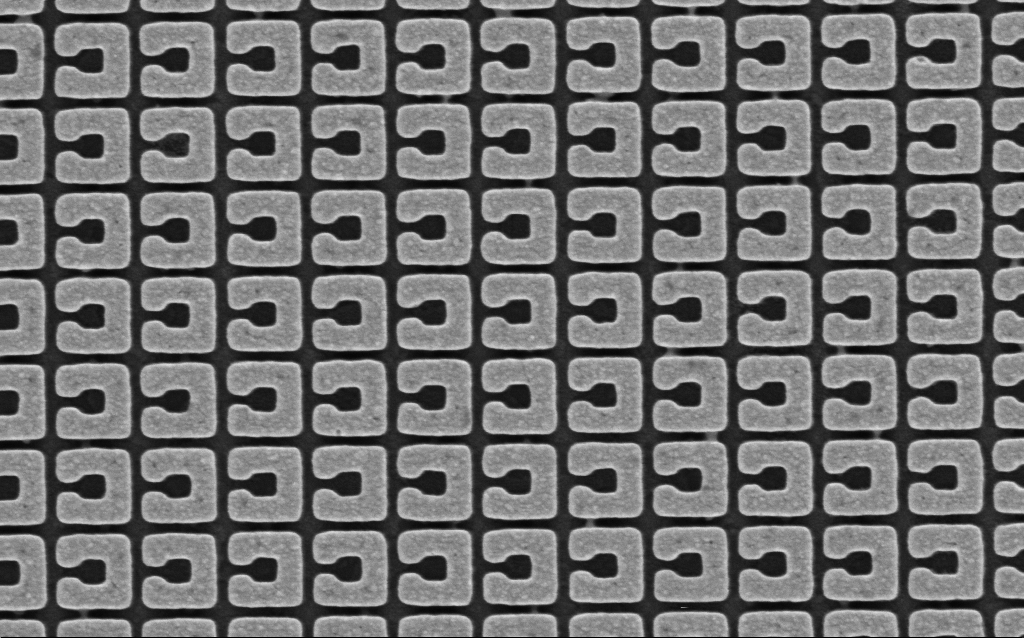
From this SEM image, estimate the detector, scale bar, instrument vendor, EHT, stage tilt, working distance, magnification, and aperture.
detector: InLens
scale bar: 1000 nm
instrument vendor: Zeiss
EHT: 3 kV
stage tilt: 0°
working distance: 4 mm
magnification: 68.08 K X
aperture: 30 µm